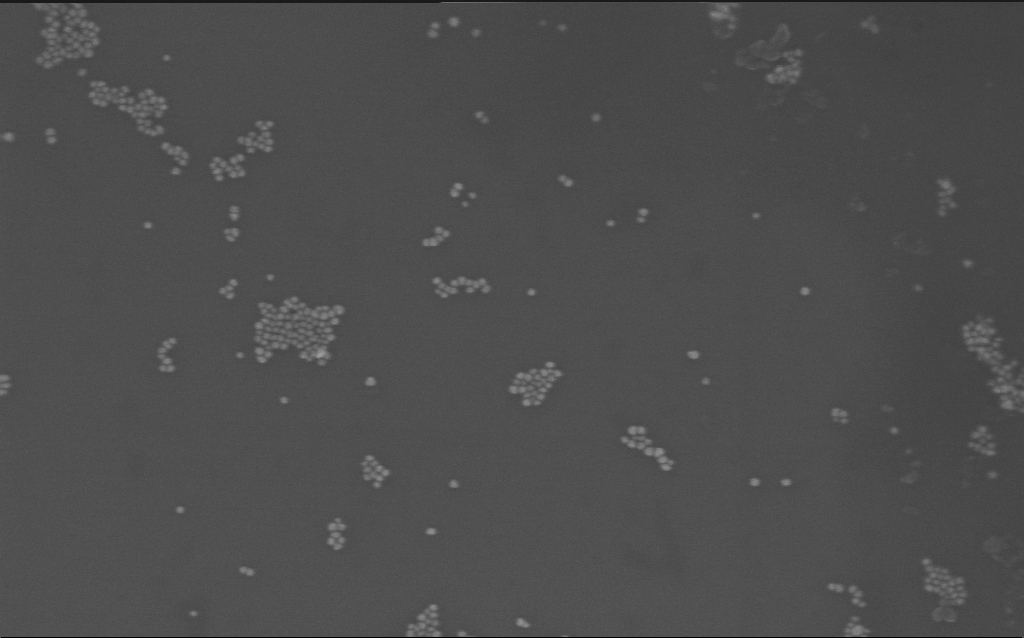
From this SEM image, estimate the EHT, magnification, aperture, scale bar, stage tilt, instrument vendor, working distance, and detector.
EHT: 10 kV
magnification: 169.96 K X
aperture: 30 µm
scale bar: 100 nm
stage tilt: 0°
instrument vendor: Zeiss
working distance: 2.9 mm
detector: InLens